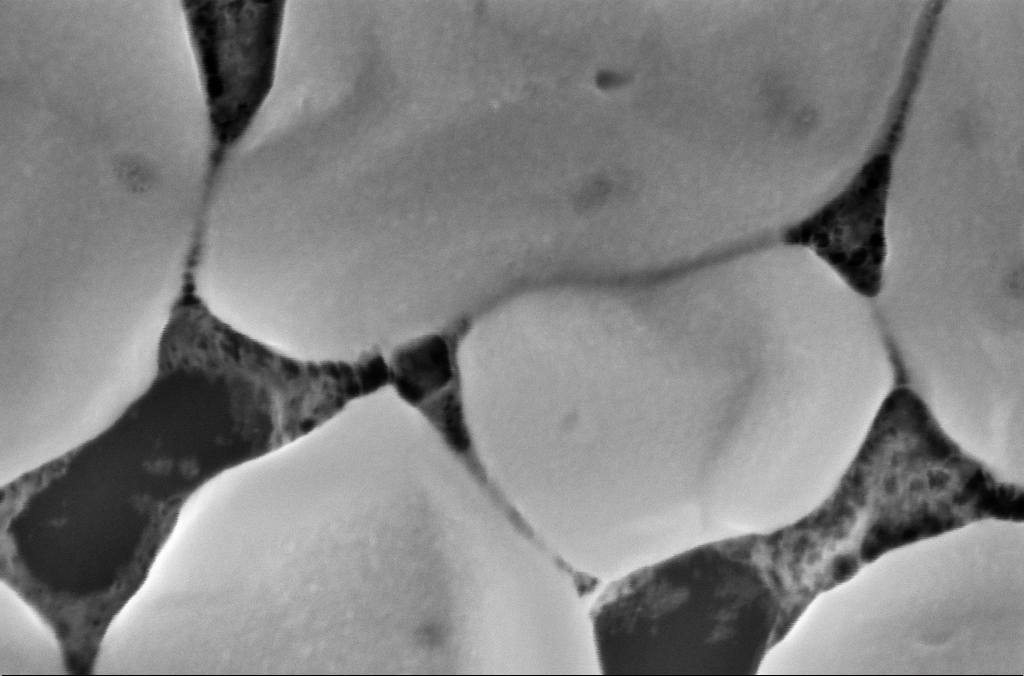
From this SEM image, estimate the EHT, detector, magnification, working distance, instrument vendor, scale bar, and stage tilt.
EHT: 5 kV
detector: InLens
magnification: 300 K X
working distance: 3 mm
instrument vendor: Zeiss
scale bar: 200 nm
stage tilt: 0°